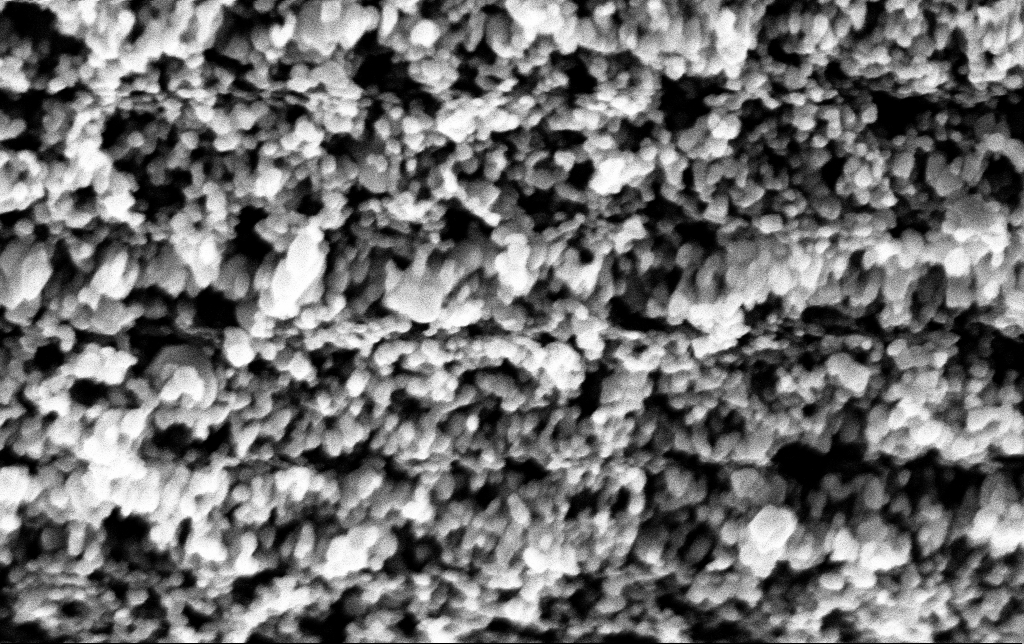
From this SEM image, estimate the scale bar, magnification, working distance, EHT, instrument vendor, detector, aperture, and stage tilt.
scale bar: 200 nm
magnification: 194.27 K X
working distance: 2.8 mm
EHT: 3 kV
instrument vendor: Zeiss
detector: InLens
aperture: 30 µm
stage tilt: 0°